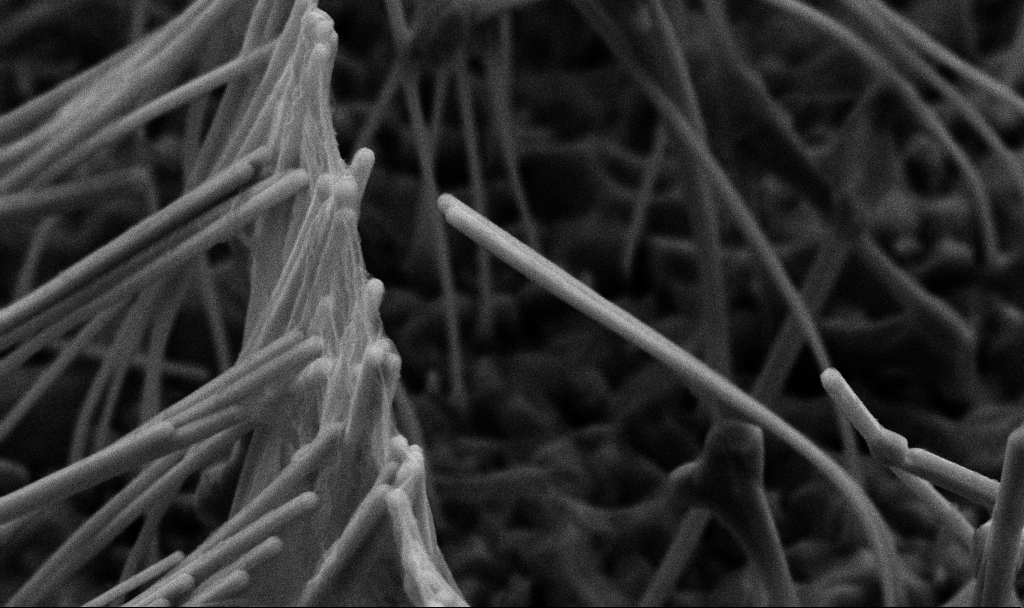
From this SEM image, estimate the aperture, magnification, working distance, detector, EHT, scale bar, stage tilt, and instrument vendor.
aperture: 30 µm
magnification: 53.7 K X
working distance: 7.2 mm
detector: SE2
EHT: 5 kV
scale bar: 1000 nm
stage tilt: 45°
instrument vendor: Zeiss